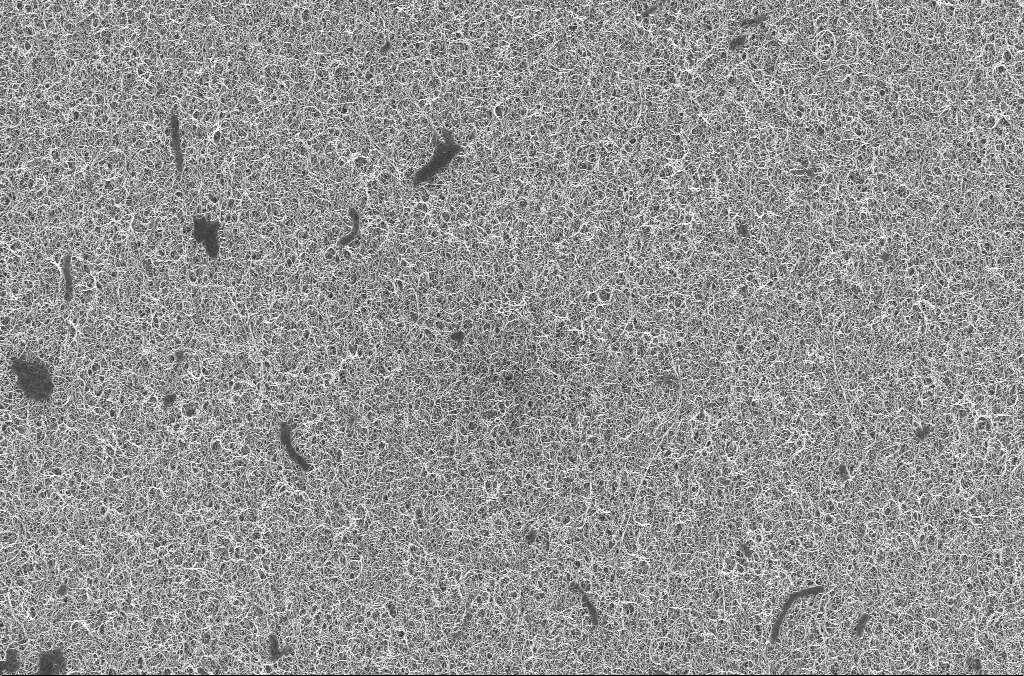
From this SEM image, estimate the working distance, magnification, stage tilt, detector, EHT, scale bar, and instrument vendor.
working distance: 4.5 mm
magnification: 5 K X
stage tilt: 0°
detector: InLens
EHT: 20 kV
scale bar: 10000 nm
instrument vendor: Zeiss